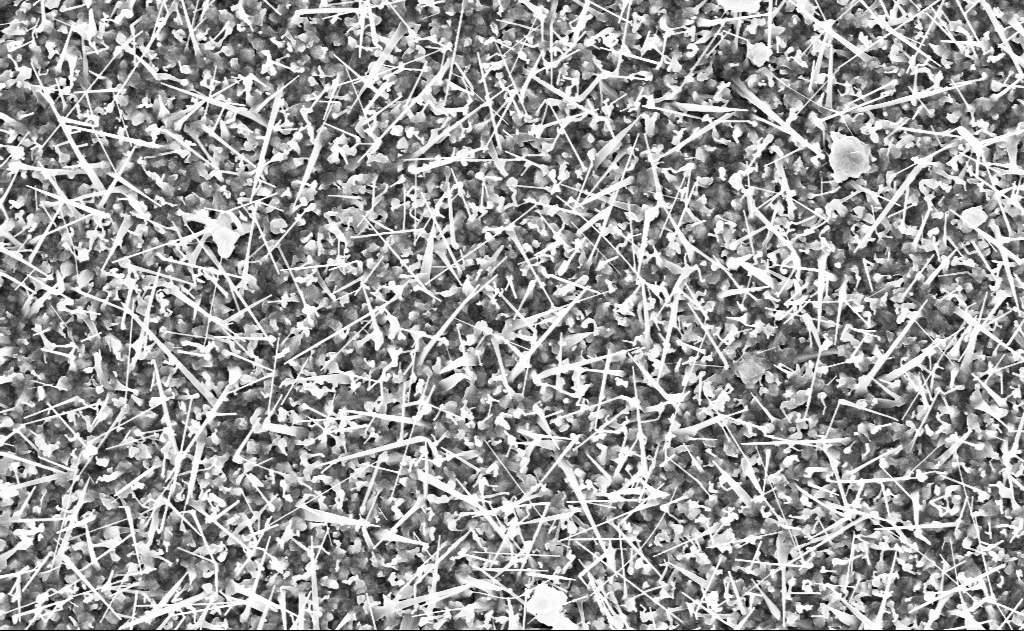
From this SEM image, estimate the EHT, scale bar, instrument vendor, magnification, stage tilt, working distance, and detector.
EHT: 10 kV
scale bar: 2000 nm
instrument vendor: Zeiss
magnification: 20 K X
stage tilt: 0°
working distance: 12 mm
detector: InLens